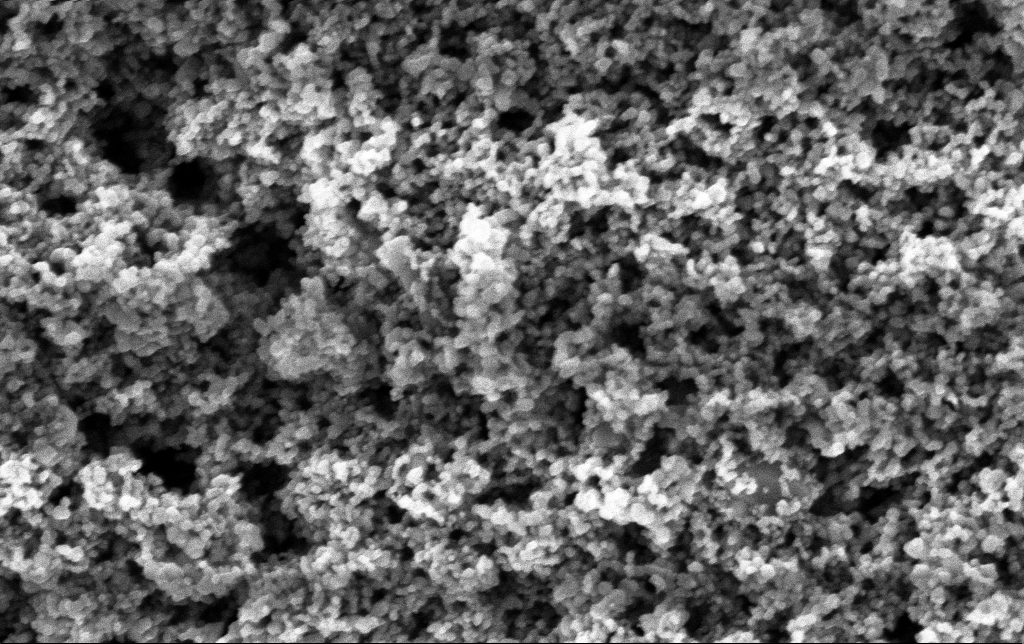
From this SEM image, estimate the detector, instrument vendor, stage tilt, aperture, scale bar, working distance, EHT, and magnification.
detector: InLens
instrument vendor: Zeiss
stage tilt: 0°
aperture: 30 µm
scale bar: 200 nm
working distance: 2.5 mm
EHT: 3 kV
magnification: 123.11 K X